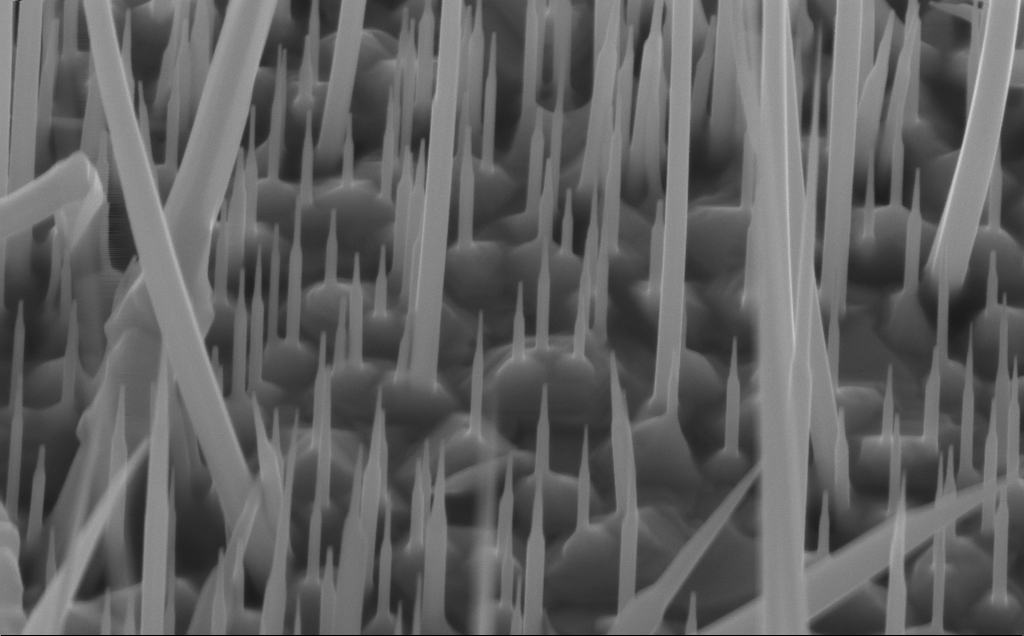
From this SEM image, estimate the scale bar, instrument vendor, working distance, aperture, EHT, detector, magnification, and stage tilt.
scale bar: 200 nm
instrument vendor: Zeiss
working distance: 4 mm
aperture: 30 µm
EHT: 10 kV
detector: InLens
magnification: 73.62 K X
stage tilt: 45°